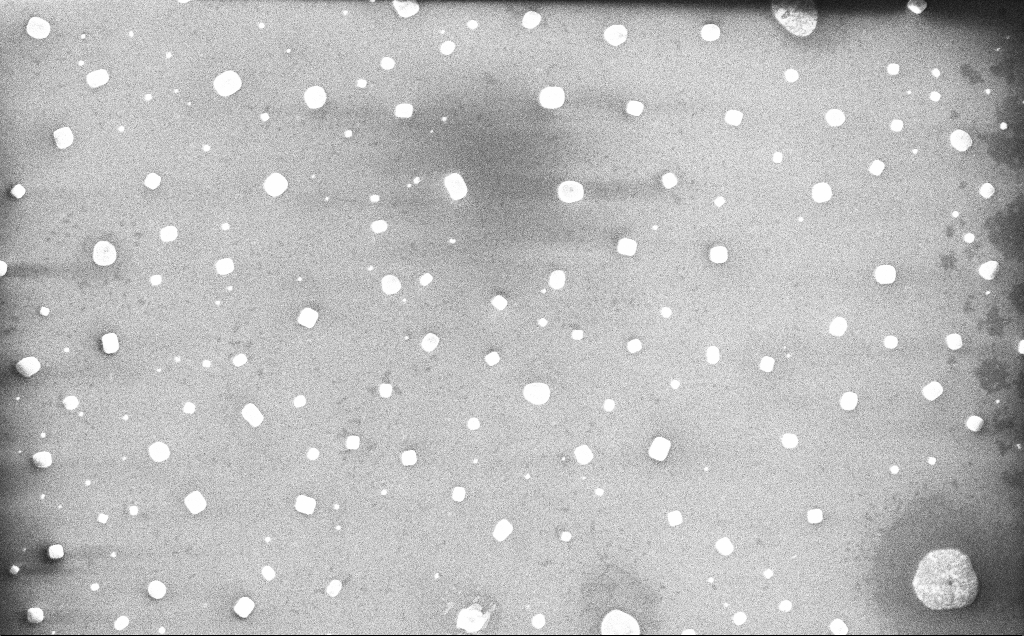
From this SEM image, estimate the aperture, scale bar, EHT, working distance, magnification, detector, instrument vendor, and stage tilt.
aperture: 30 µm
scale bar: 1000 nm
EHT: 10 kV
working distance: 5 mm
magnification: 21.89 K X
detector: InLens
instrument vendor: Zeiss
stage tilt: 0°